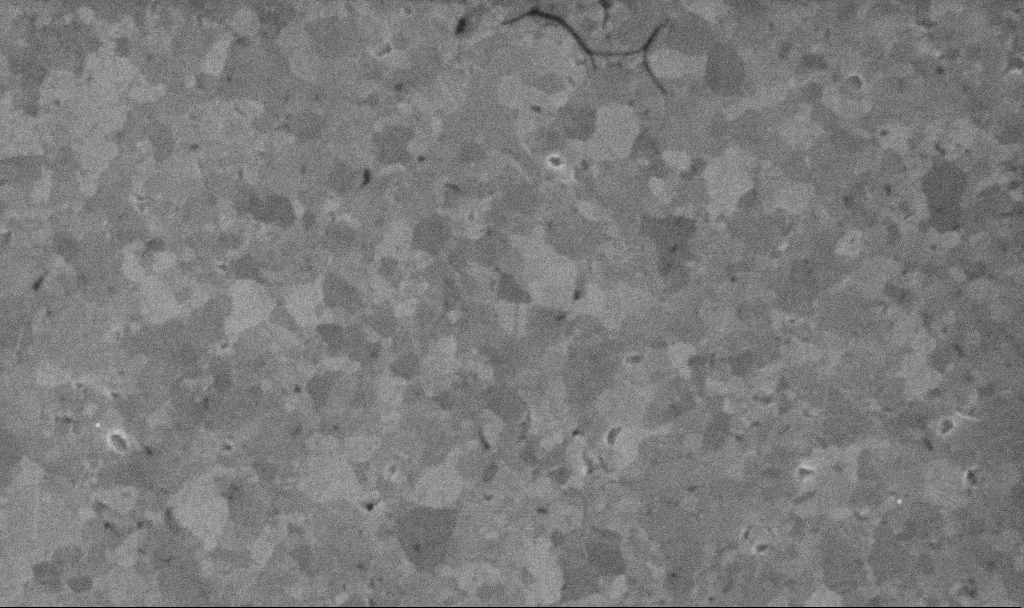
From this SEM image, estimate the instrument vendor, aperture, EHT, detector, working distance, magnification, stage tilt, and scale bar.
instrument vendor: Zeiss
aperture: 30 µm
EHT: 10 kV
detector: InLens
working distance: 3.1 mm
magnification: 46.56 K X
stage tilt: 0°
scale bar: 1000 nm